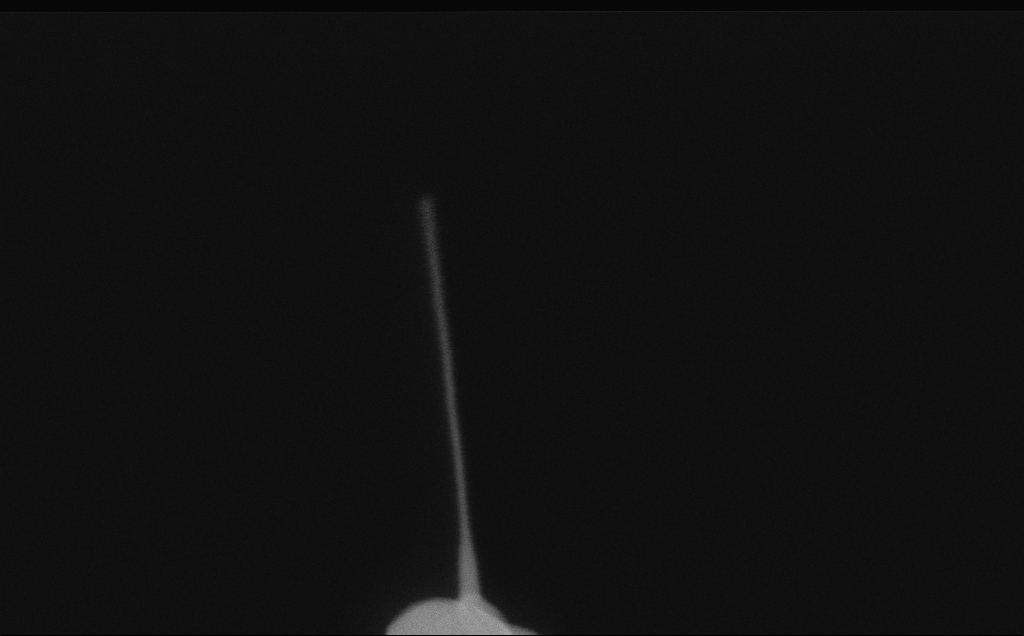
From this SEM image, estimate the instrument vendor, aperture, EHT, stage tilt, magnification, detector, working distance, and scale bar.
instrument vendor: Zeiss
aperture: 30 µm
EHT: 10 kV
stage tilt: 0°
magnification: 257.27 K X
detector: InLens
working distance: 6 mm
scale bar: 200 nm